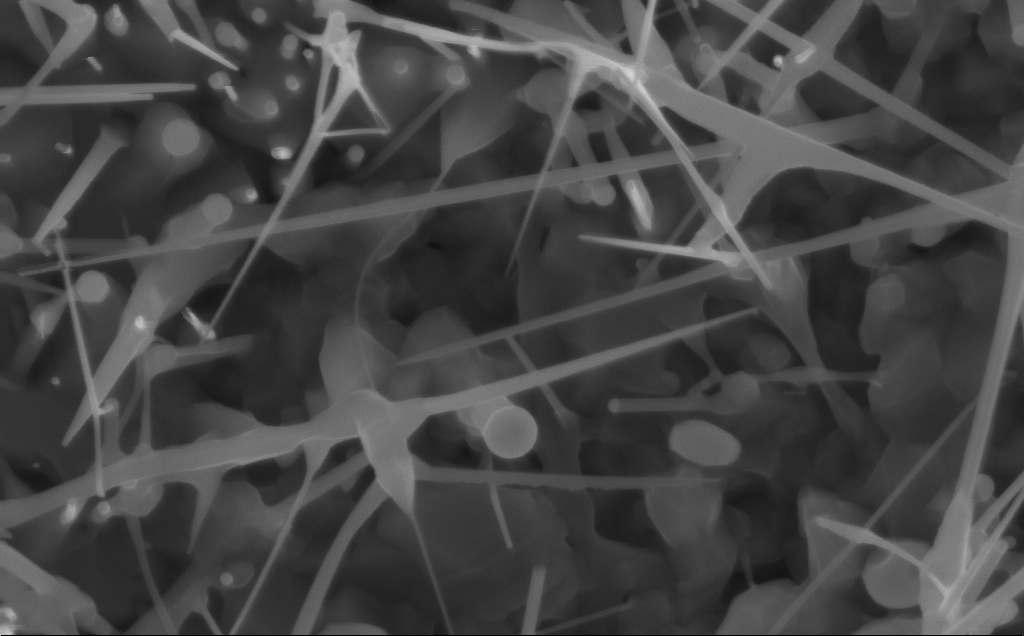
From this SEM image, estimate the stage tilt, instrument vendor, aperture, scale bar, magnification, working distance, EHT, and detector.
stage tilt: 0°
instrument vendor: Zeiss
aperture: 30 µm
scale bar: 200 nm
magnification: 80 K X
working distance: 3 mm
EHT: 10 kV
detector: InLens